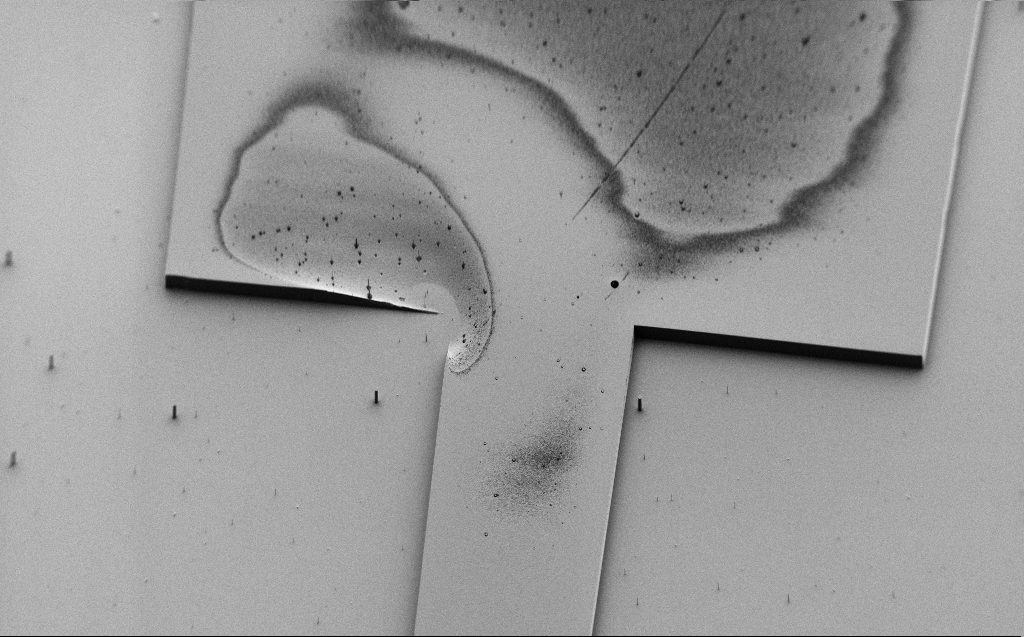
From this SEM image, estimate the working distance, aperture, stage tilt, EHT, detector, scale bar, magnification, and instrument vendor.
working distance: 7 mm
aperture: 30 µm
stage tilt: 45°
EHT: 1.1 kV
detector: SE2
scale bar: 100000 nm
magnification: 0.343 K X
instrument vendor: Zeiss